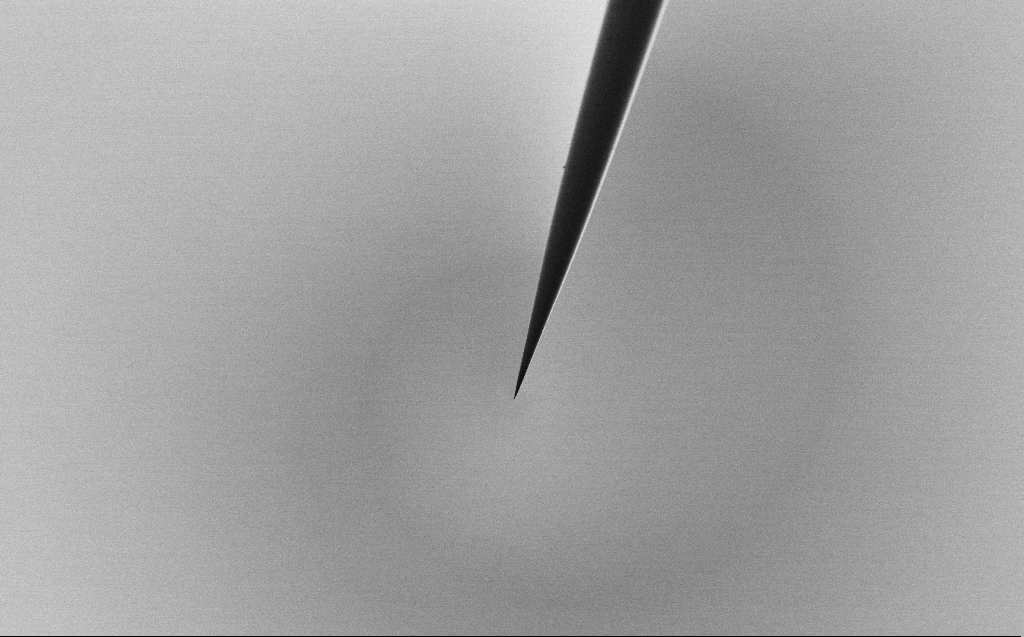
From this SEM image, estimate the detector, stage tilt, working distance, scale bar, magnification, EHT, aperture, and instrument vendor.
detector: SE2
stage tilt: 45°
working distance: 5 mm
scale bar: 20000 nm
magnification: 1 K X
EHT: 1 kV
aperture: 30 µm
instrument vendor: Zeiss